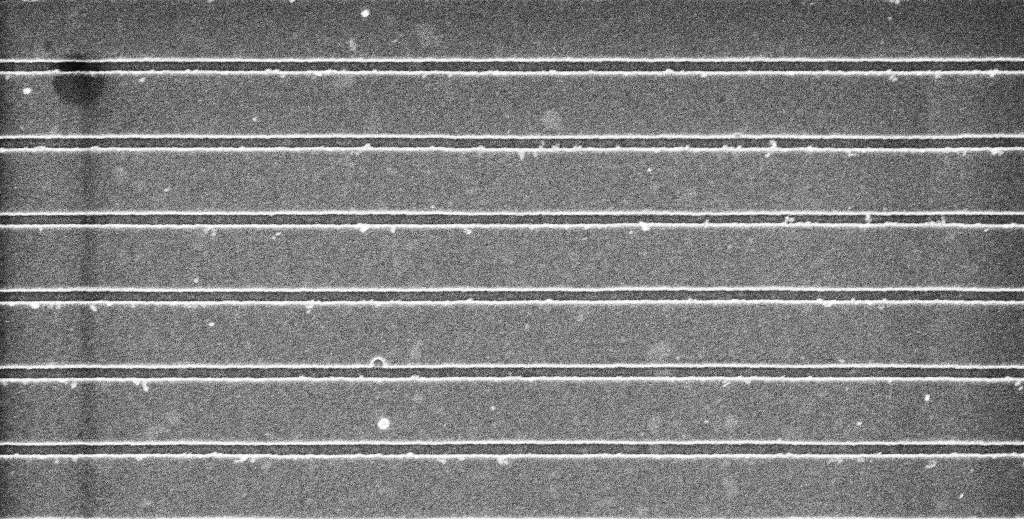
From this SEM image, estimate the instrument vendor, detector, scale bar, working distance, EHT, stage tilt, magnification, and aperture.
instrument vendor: Zeiss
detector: InLens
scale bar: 1000 nm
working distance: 5.3 mm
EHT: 5 kV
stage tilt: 0°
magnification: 31.33 K X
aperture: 30 µm